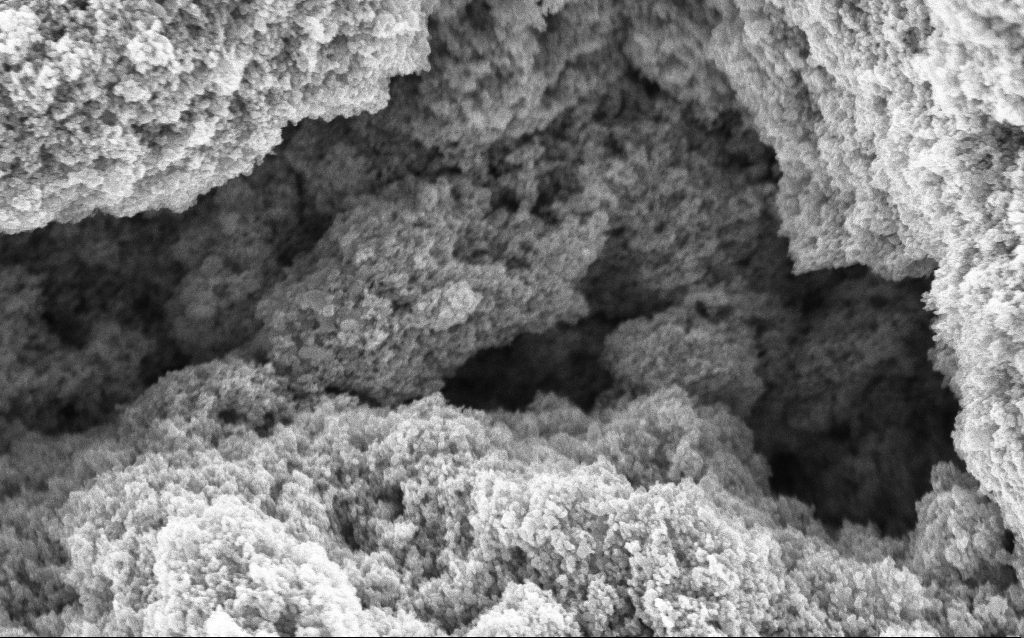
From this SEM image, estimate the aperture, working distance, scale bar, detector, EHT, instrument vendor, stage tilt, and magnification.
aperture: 30 µm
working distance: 4.6 mm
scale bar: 1000 nm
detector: InLens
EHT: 5 kV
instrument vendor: Zeiss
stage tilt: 0°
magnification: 68.7 K X